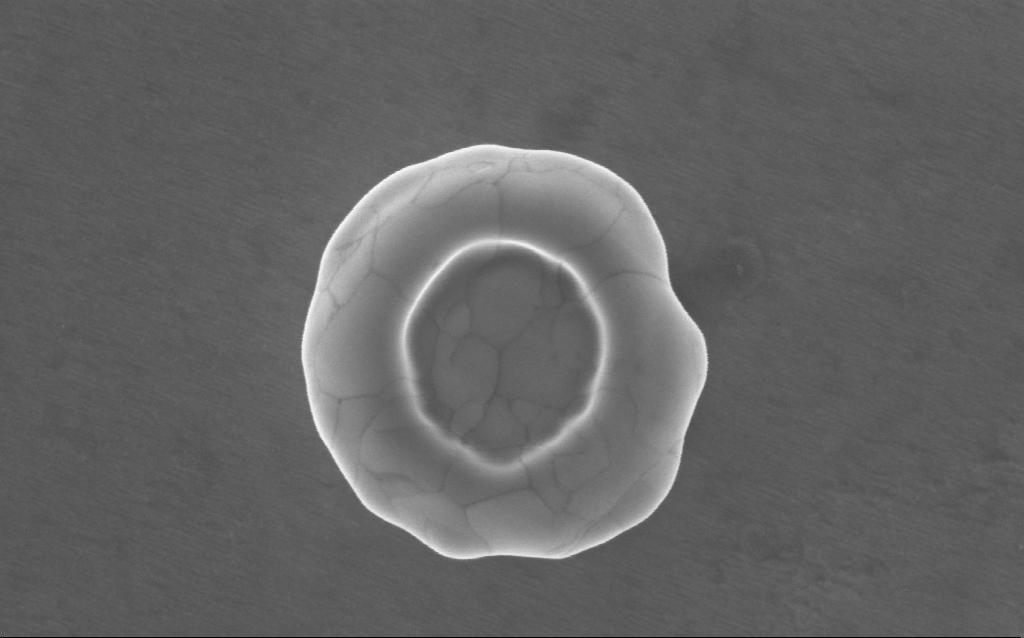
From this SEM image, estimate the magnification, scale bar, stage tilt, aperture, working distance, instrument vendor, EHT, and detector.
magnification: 152 K X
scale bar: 100 nm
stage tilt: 0°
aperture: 30 µm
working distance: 3 mm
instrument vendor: Zeiss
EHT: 5 kV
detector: InLens